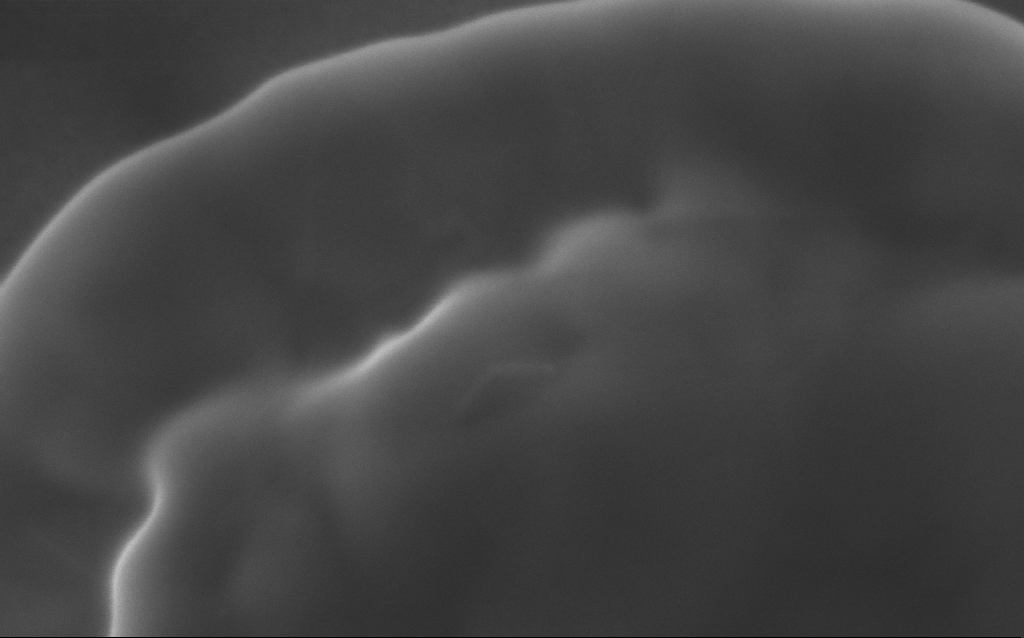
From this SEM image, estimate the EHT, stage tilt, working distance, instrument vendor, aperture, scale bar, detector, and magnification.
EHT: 5 kV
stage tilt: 0°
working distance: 3 mm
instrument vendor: Zeiss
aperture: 30 µm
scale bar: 100 nm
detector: InLens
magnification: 413.44 K X